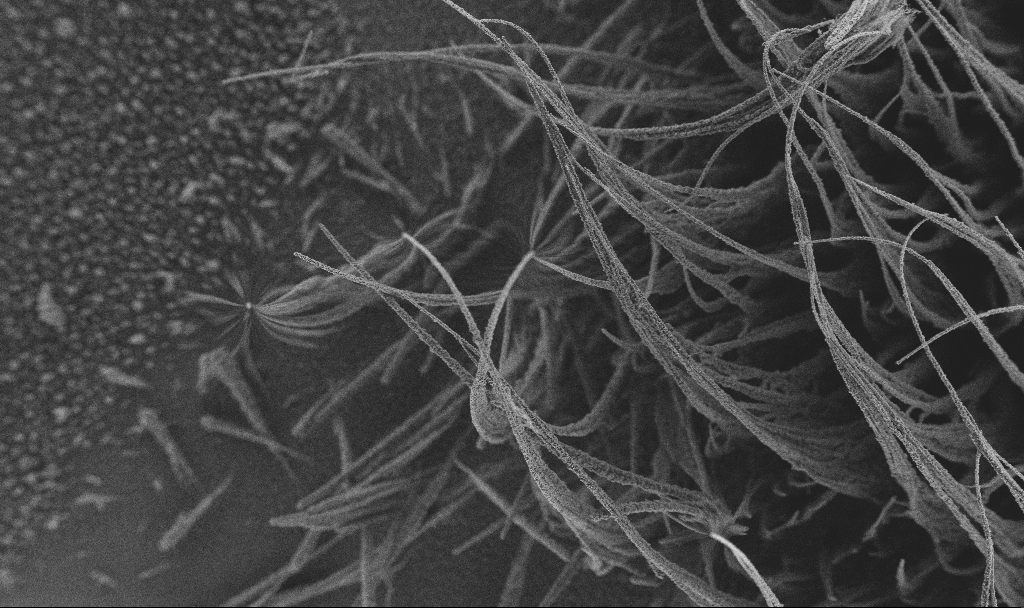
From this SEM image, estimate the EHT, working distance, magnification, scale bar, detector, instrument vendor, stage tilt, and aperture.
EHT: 3 kV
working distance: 2.9 mm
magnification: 0.15 K X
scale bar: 100000 nm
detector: SE2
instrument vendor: Zeiss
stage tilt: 0°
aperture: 30 µm